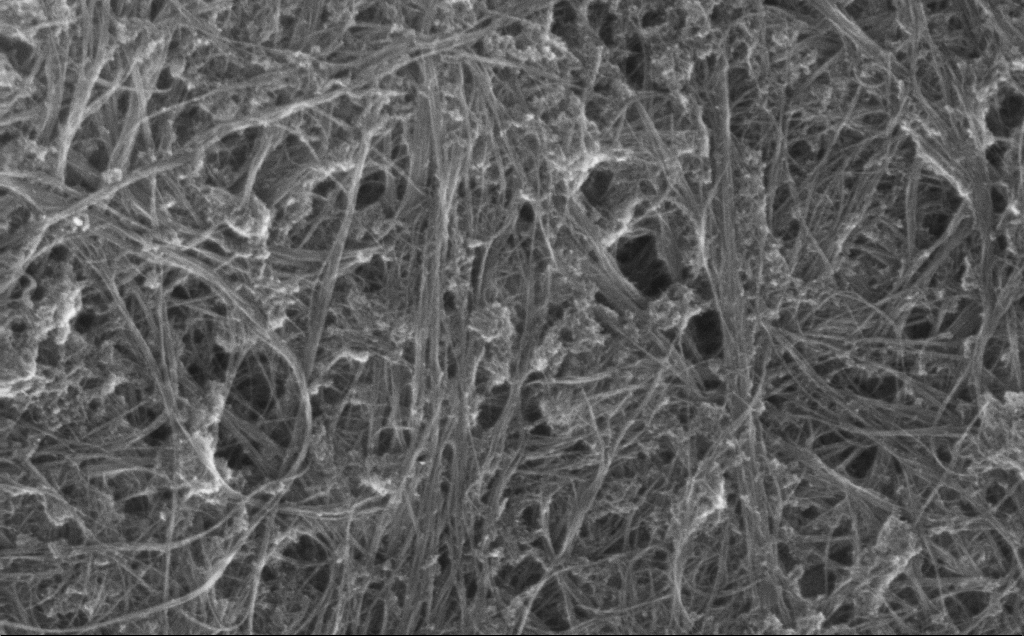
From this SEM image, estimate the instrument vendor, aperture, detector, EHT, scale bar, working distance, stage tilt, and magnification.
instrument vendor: Zeiss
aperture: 30 µm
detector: InLens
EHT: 10 kV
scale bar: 1000 nm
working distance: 3 mm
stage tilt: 0°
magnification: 70.52 K X